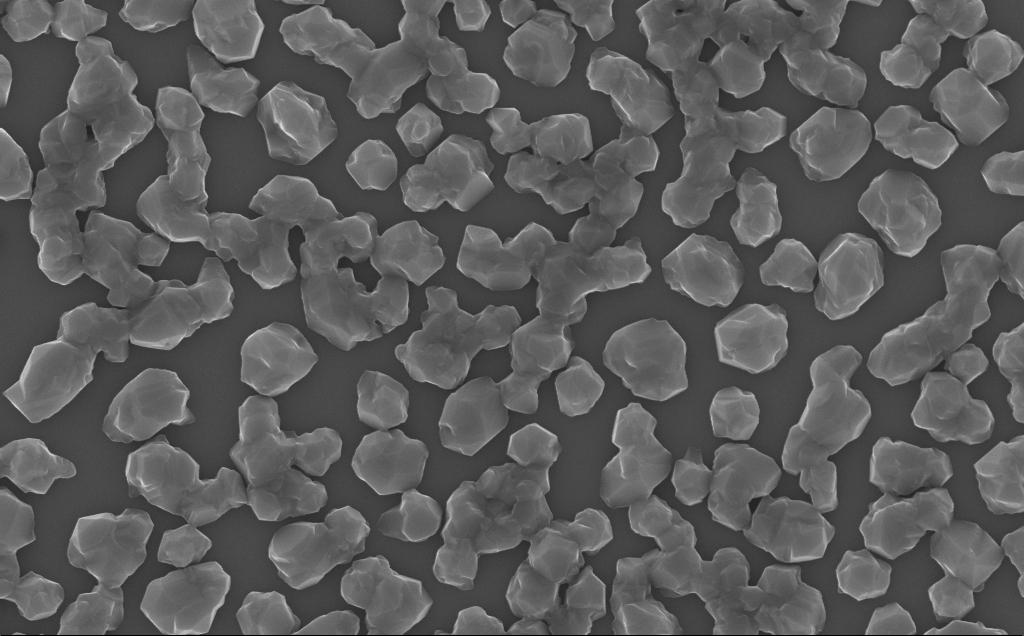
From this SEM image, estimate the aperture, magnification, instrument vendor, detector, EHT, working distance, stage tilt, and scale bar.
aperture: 30 µm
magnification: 40 K X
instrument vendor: Zeiss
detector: InLens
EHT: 10 kV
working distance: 6 mm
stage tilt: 0°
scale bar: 1000 nm